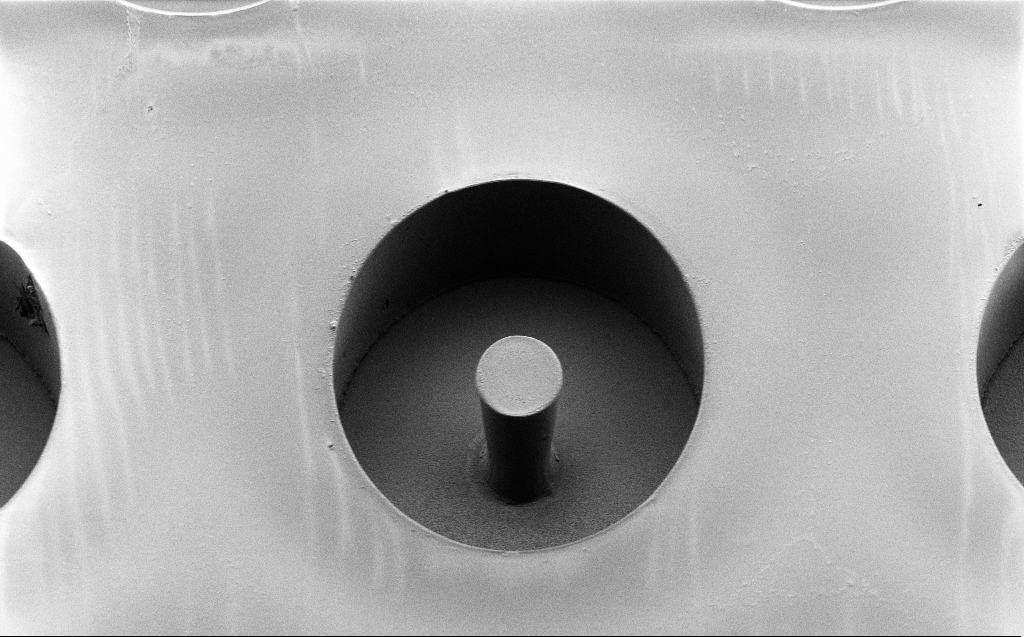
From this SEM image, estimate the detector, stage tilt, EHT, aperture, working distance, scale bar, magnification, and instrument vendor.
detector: SE2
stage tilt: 45°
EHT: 2 kV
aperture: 30 µm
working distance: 7 mm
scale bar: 20000 nm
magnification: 1.49 K X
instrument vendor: Zeiss